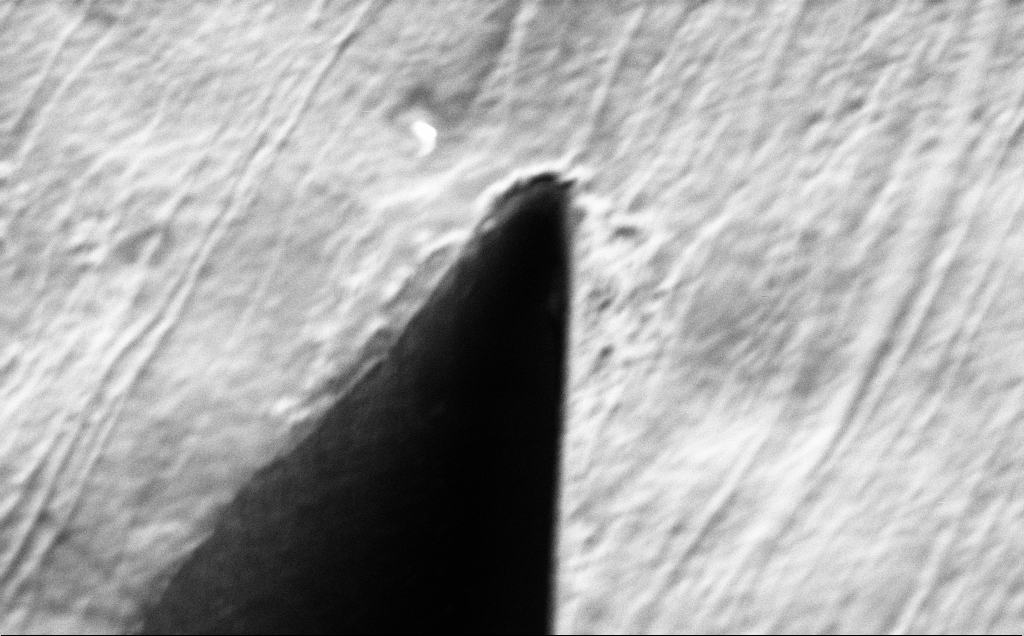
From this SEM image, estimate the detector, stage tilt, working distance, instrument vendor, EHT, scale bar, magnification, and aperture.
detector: SE2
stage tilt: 45°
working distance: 8 mm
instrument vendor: Zeiss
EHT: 1.2 kV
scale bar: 2000 nm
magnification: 22.38 K X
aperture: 30 µm